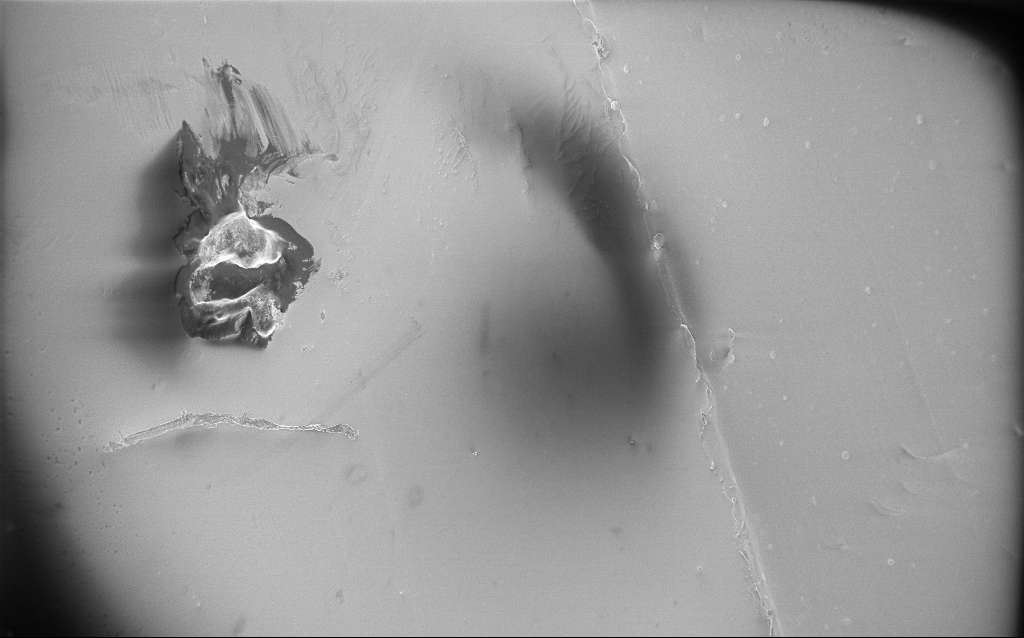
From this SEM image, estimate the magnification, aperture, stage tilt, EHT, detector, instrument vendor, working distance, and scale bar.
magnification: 0.11 K X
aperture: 30 µm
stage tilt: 0°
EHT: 3 kV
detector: InLens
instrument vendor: Zeiss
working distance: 2.7 mm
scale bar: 200000 nm